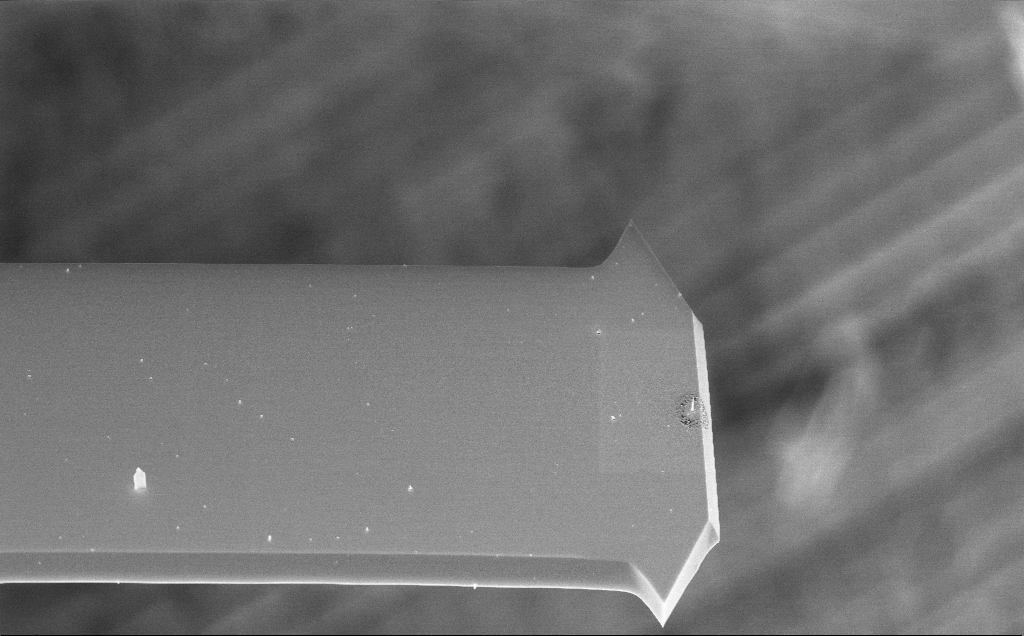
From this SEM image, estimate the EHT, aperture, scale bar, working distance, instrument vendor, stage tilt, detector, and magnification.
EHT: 10 kV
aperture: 30 µm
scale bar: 10000 nm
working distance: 3 mm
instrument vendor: Zeiss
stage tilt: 44.2°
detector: InLens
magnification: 3.16 K X